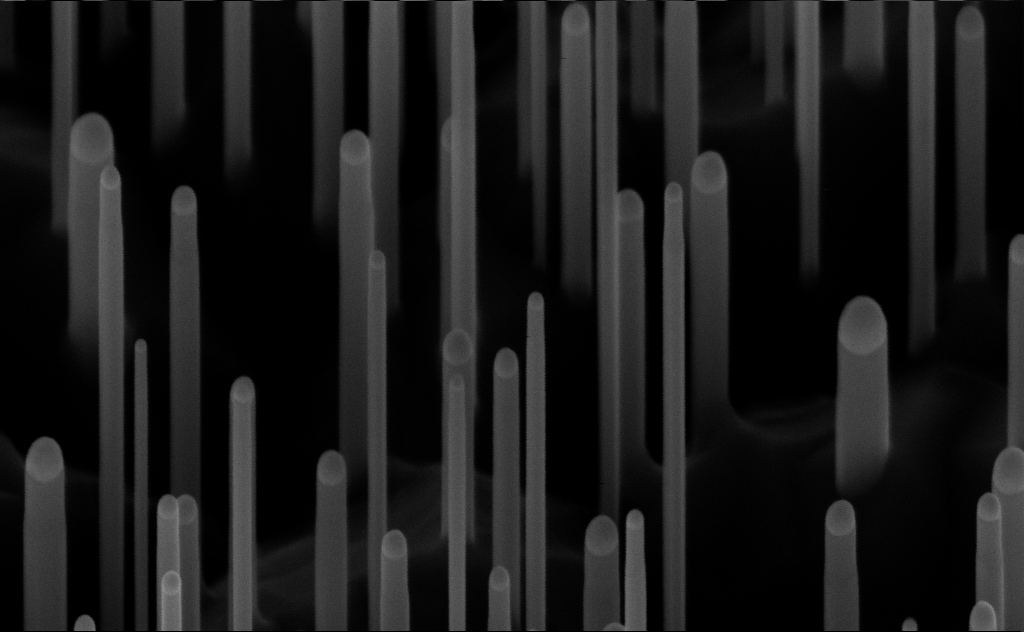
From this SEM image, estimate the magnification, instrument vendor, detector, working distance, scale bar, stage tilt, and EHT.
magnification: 150 K X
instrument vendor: Zeiss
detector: InLens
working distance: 7 mm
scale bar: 200 nm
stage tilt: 45°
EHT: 10 kV